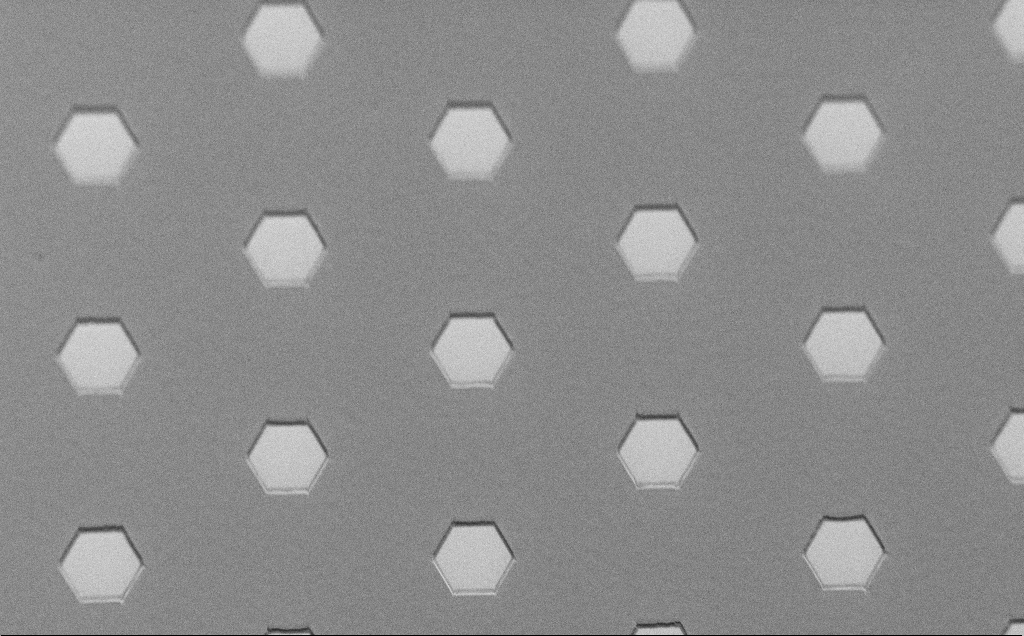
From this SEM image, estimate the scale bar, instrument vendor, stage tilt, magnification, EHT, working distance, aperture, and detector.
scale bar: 100000 nm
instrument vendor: Zeiss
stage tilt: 45°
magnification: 0.523 K X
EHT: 1.5 kV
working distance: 7 mm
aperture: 30 µm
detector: SE2